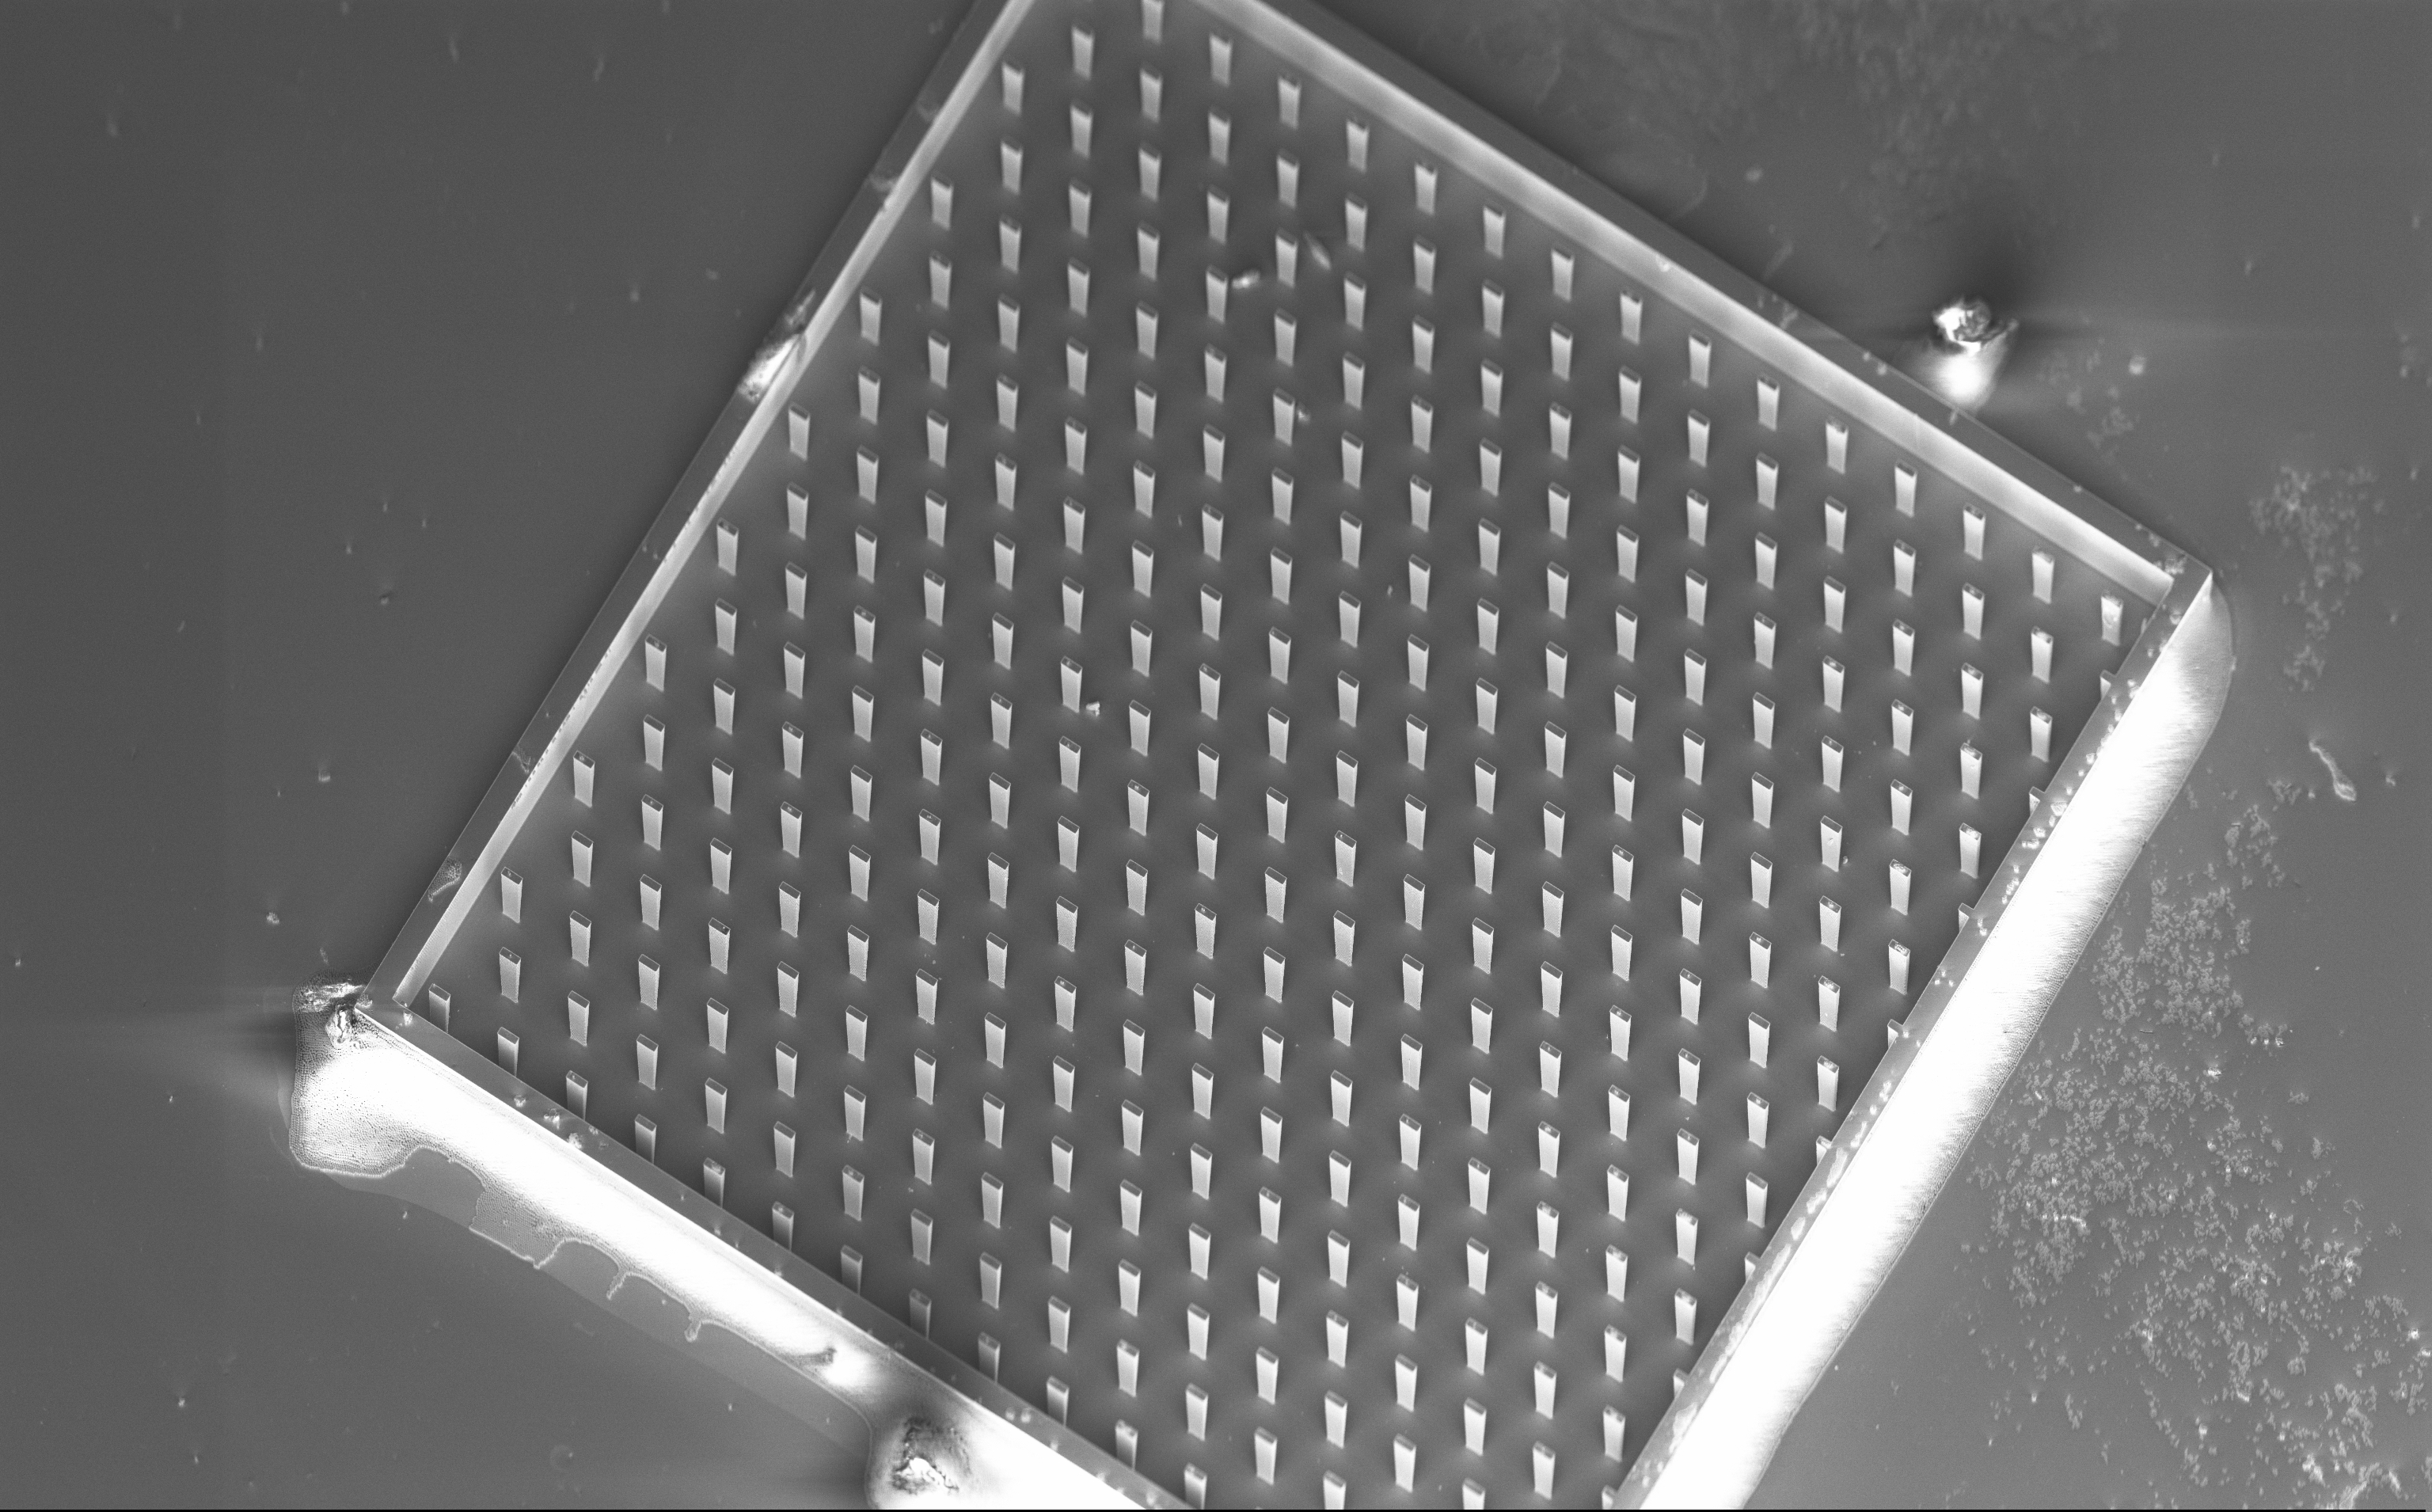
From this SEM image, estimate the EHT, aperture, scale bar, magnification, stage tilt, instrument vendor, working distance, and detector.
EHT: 5 kV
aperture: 30 µm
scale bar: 100000 nm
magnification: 0.44 K X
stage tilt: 45°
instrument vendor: Zeiss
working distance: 7 mm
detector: InLens